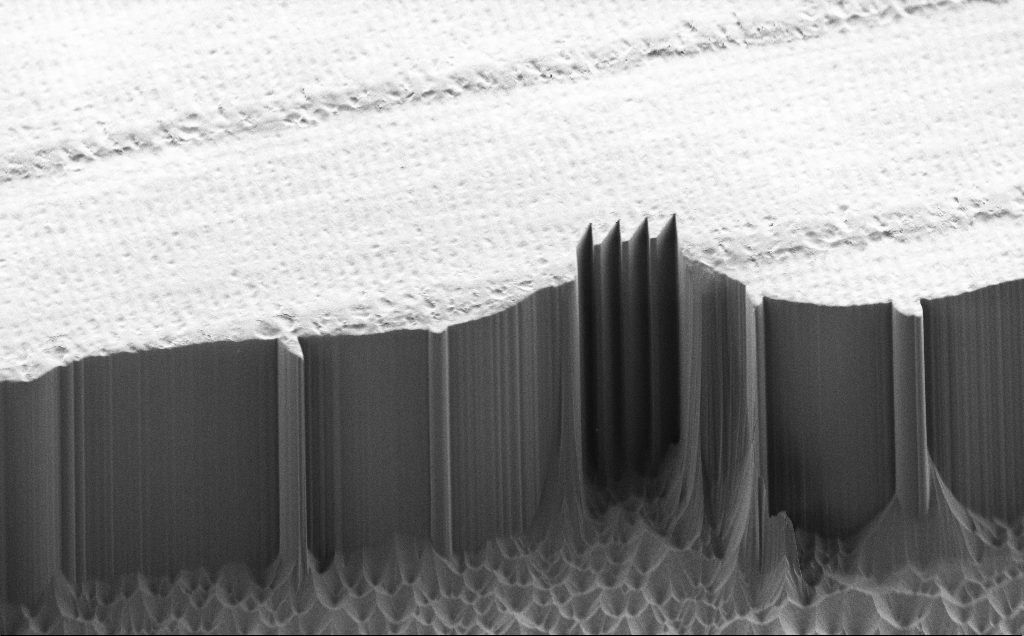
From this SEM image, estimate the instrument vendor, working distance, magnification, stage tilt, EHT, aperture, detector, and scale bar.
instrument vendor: Zeiss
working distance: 7 mm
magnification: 0.89 K X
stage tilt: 45°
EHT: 1.2 kV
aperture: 30 µm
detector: SE2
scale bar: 20000 nm